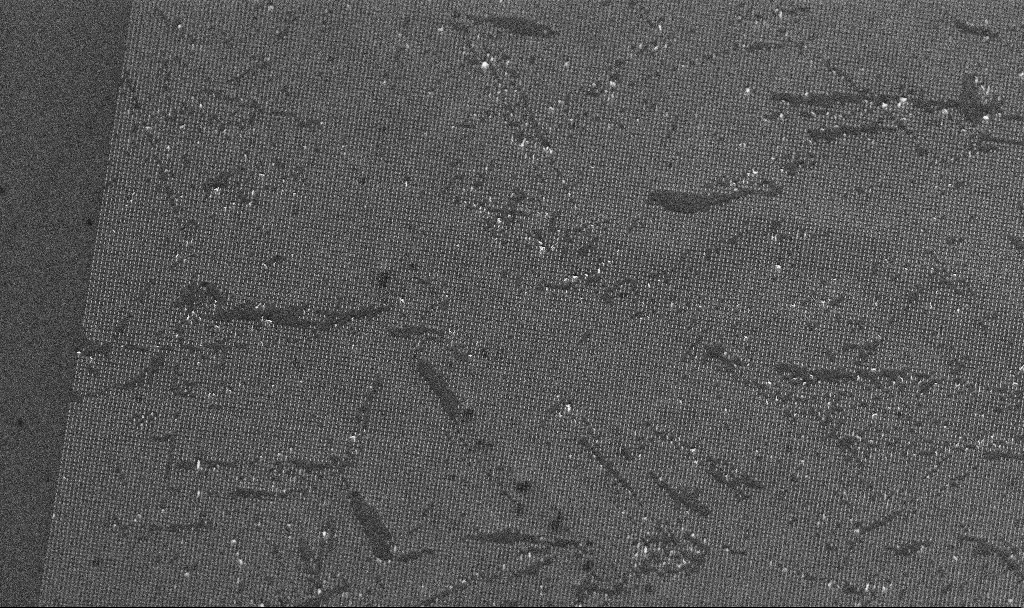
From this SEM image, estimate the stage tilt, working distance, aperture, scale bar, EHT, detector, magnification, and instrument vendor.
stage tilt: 45°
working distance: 7.4 mm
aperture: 30 µm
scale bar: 10000 nm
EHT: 5 kV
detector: InLens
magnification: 6.51 K X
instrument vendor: Zeiss